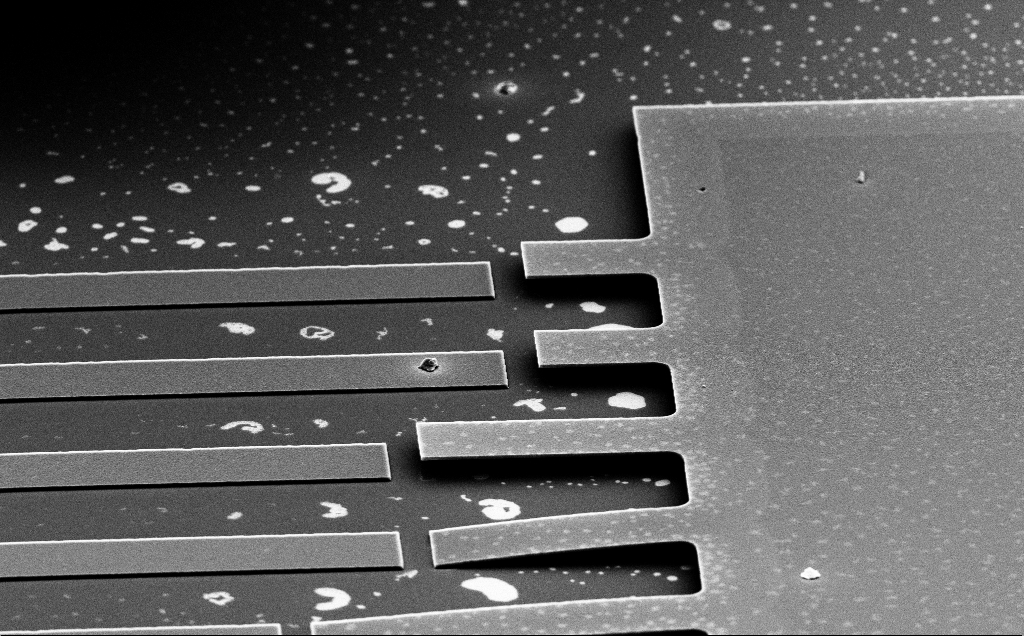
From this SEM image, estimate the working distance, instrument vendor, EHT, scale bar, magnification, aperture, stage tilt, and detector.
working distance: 18 mm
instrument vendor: Zeiss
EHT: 10 kV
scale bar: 20000 nm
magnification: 1.91 K X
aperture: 30 µm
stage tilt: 65.4°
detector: SE2